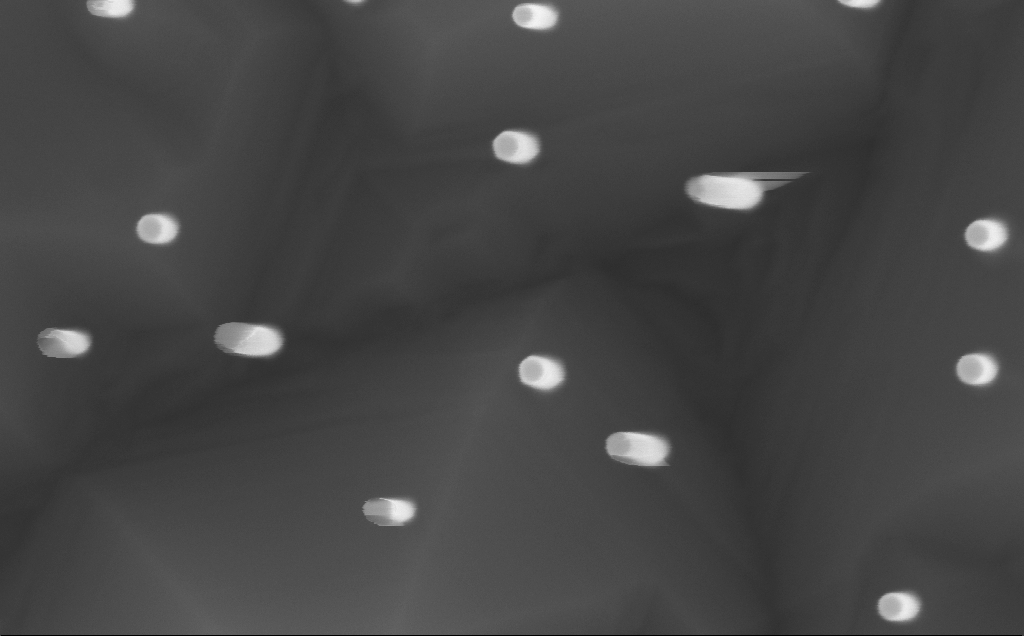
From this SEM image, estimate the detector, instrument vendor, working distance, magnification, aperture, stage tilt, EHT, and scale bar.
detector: InLens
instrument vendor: Zeiss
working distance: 7 mm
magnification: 211.12 K X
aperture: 30 µm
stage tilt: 0.2°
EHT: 10 kV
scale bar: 100 nm